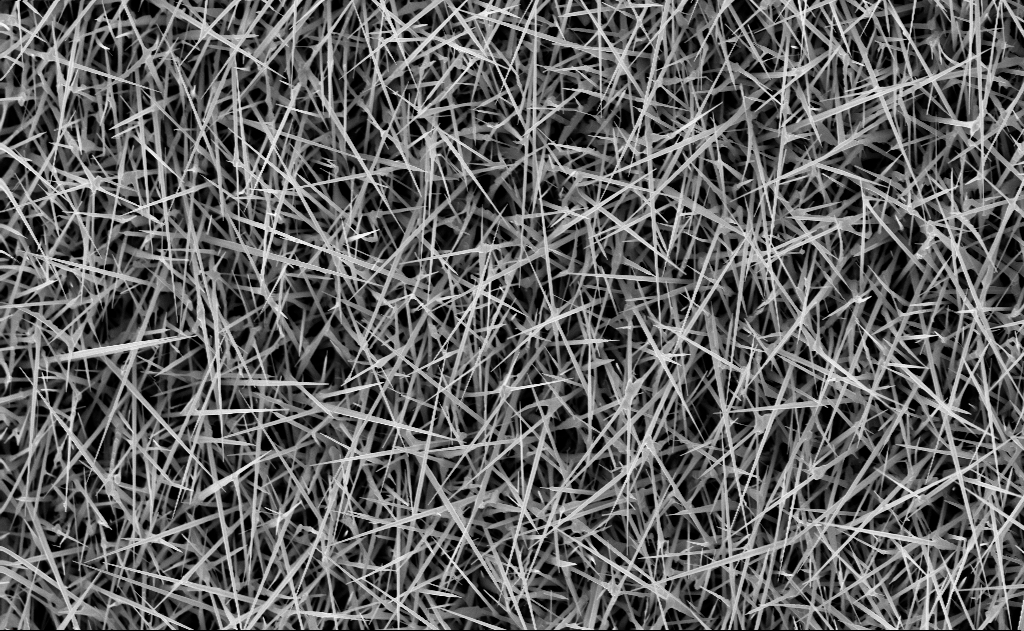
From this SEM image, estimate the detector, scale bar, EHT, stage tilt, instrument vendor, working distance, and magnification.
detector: InLens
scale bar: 2000 nm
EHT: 10 kV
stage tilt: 0°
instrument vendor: Zeiss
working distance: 10 mm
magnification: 10 K X